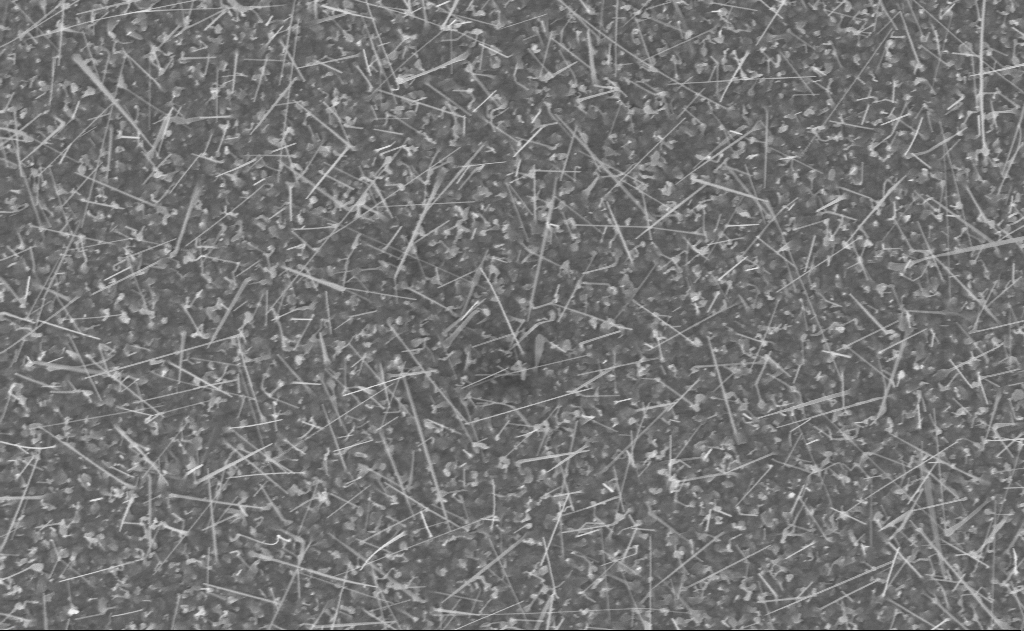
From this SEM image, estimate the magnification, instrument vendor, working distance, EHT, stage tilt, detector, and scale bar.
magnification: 20 K X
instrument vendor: Zeiss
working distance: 20 mm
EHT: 10 kV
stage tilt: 0°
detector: InLens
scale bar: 1000 nm